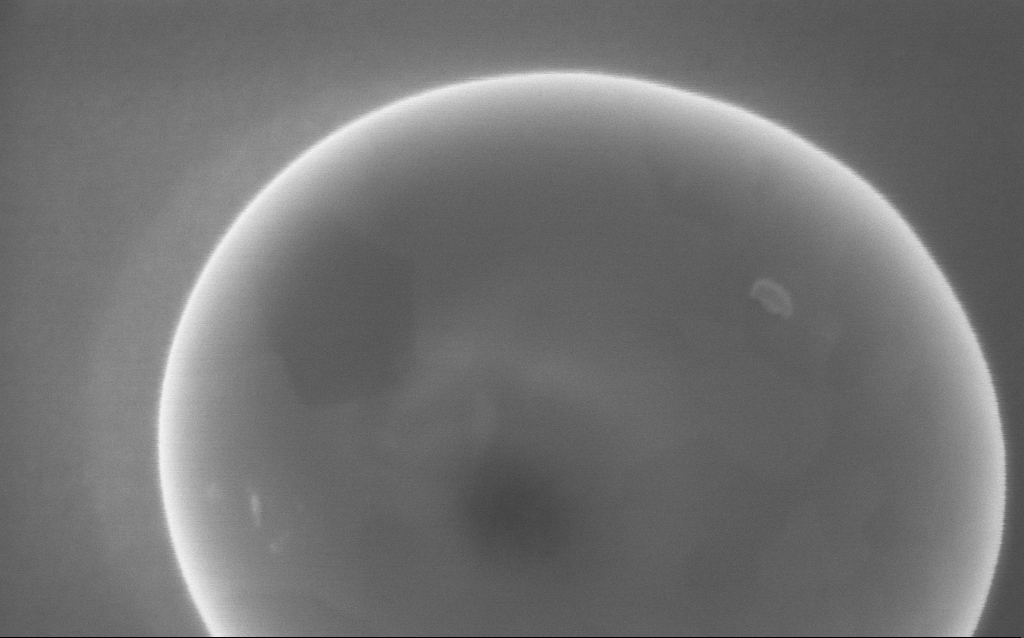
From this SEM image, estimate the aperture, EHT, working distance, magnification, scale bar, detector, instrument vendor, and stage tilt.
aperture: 30 µm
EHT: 5 kV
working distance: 3 mm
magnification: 180 K X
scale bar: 200 nm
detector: InLens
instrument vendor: Zeiss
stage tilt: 0°